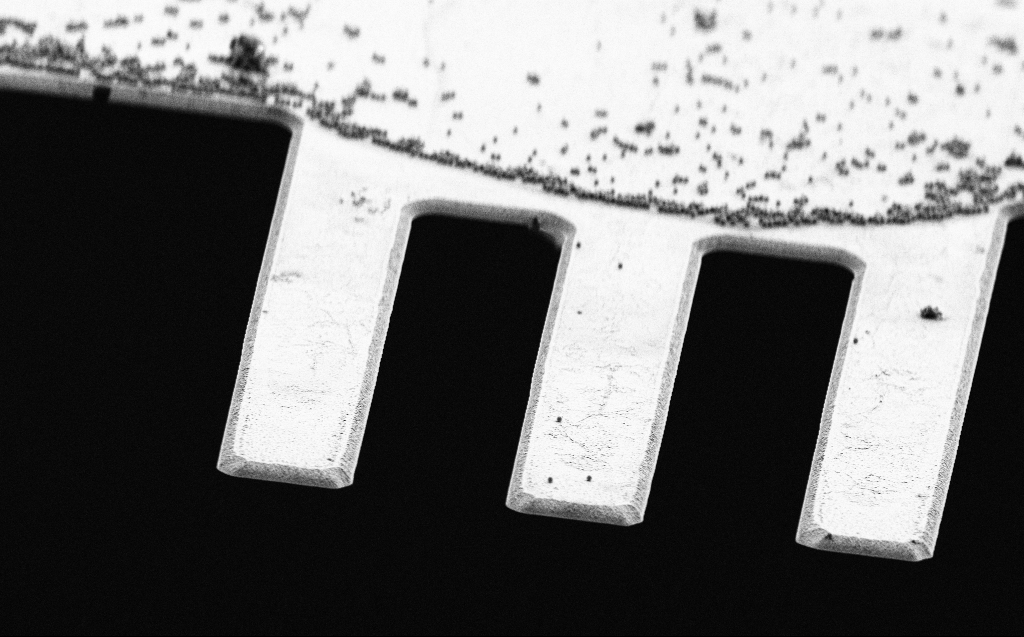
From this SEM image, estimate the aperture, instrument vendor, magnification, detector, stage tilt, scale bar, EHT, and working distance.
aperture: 30 µm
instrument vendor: Zeiss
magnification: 2.7 K X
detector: SE2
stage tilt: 64.8°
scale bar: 10000 nm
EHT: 3 kV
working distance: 7 mm